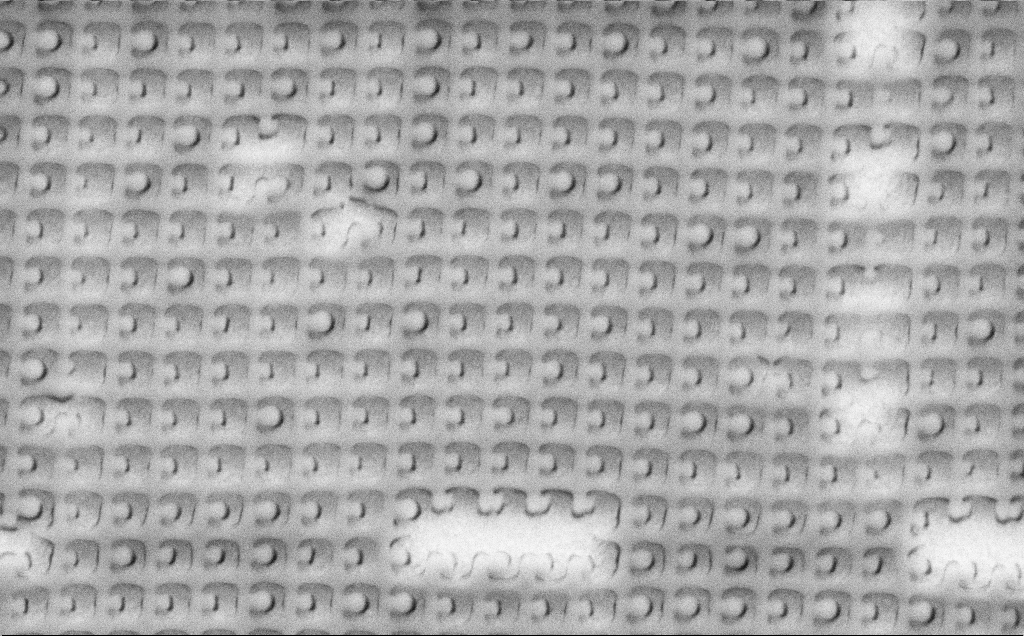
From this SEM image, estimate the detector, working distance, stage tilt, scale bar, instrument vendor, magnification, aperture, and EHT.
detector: SE2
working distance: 8 mm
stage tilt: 0°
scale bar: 1000 nm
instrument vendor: Zeiss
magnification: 37.12 K X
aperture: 30 µm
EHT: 3 kV